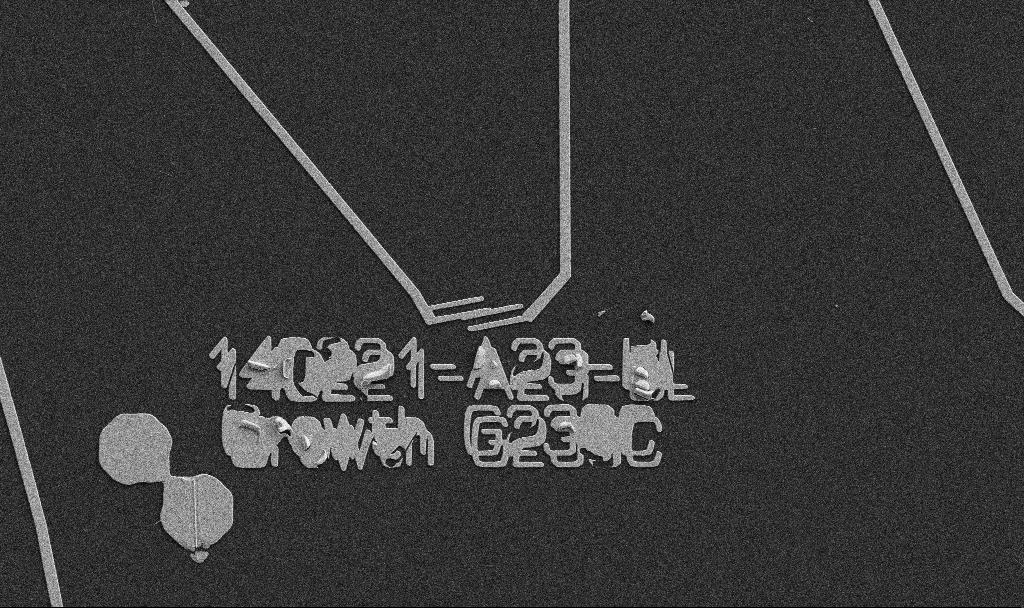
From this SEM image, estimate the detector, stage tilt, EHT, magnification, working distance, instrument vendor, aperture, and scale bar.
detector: SE2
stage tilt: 0°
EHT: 5 kV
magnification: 4.55 K X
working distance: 10.7 mm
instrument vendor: Zeiss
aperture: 30 µm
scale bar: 10000 nm